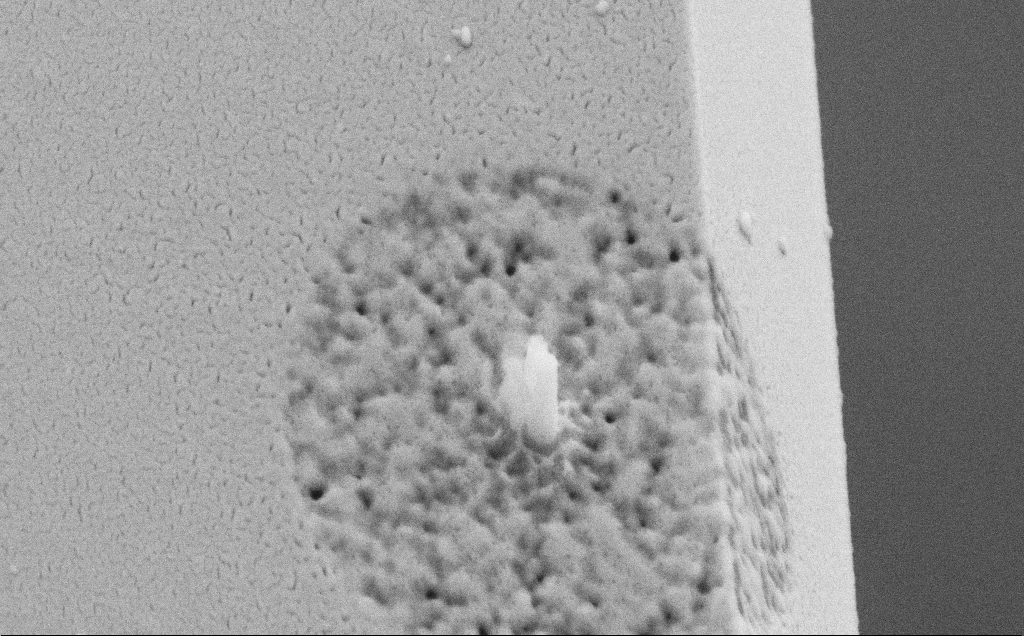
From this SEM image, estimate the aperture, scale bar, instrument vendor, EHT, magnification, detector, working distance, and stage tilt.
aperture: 30 µm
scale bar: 1000 nm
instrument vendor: Zeiss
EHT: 10 kV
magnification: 38.19 K X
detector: SE2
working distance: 3 mm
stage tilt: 44.2°